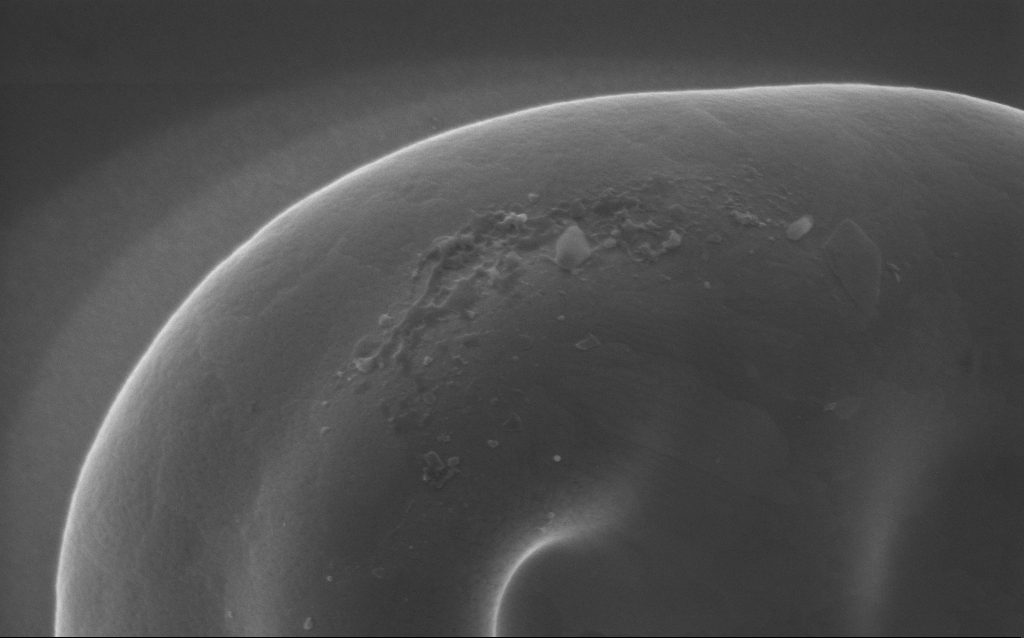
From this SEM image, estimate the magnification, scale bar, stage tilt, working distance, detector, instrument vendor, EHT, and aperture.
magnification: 100 K X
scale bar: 200 nm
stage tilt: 0°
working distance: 3 mm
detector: InLens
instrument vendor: Zeiss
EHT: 5 kV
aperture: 30 µm